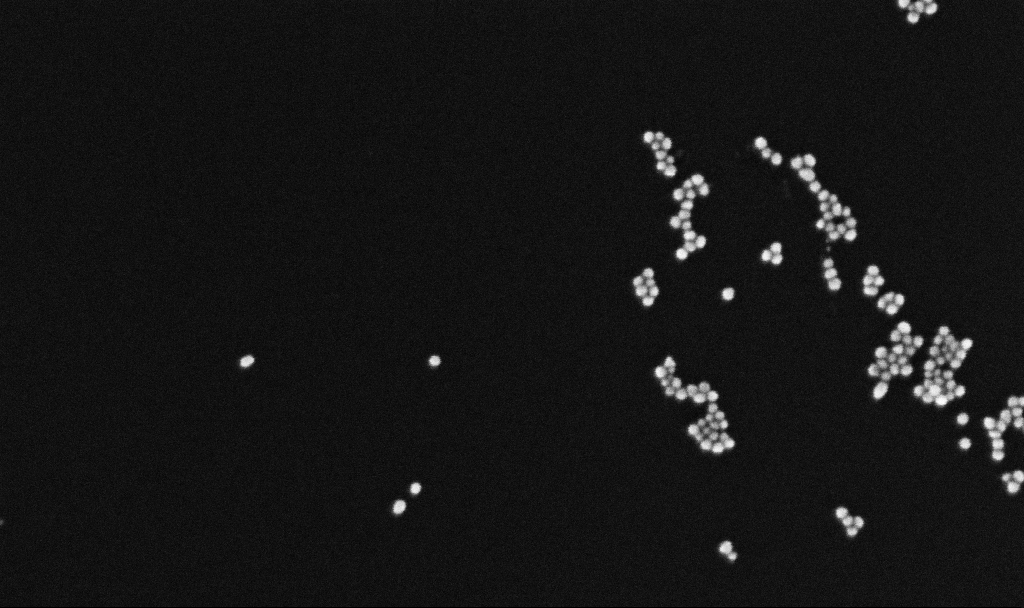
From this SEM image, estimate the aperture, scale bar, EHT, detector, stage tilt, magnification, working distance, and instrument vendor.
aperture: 30 µm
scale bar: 100 nm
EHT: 10 kV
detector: InLens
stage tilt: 0°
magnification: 300 K X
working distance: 3.4 mm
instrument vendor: Zeiss